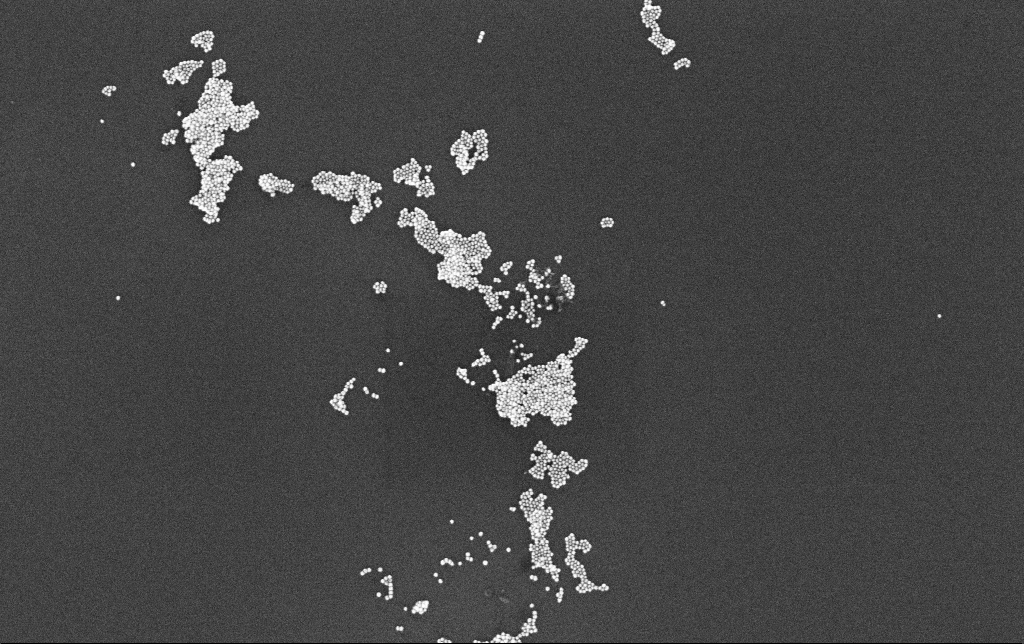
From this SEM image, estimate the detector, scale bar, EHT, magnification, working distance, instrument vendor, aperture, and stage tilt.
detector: InLens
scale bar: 200 nm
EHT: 10 kV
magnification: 100 K X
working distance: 3.4 mm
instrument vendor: Zeiss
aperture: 30 µm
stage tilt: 0°